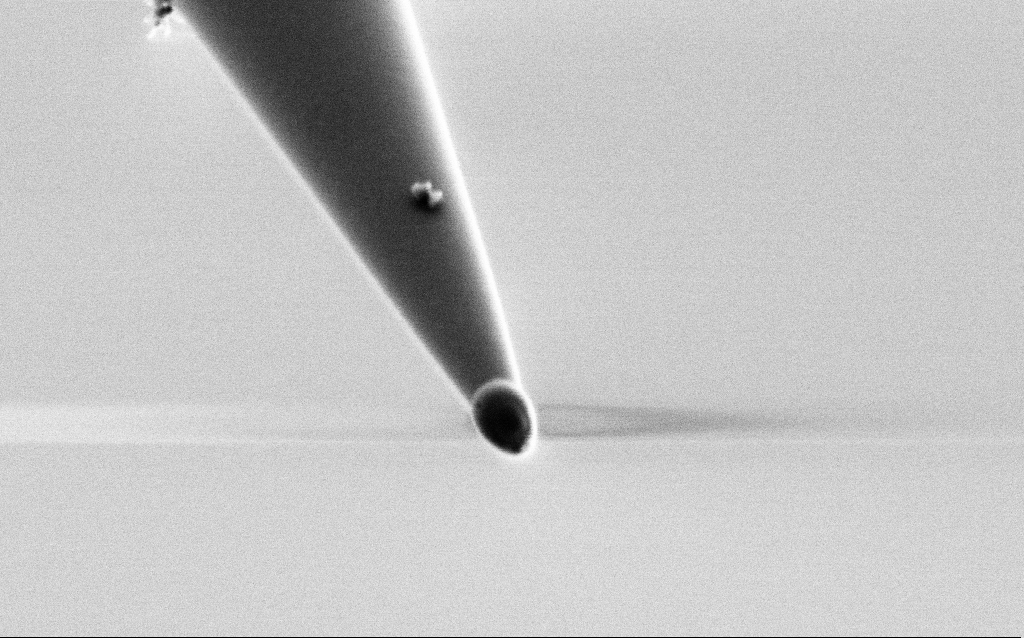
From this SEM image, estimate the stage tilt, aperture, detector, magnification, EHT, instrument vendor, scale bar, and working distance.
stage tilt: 45°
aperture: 30 µm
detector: SE2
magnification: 100 K X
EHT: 1 kV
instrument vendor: Zeiss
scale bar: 200 nm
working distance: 6.6 mm